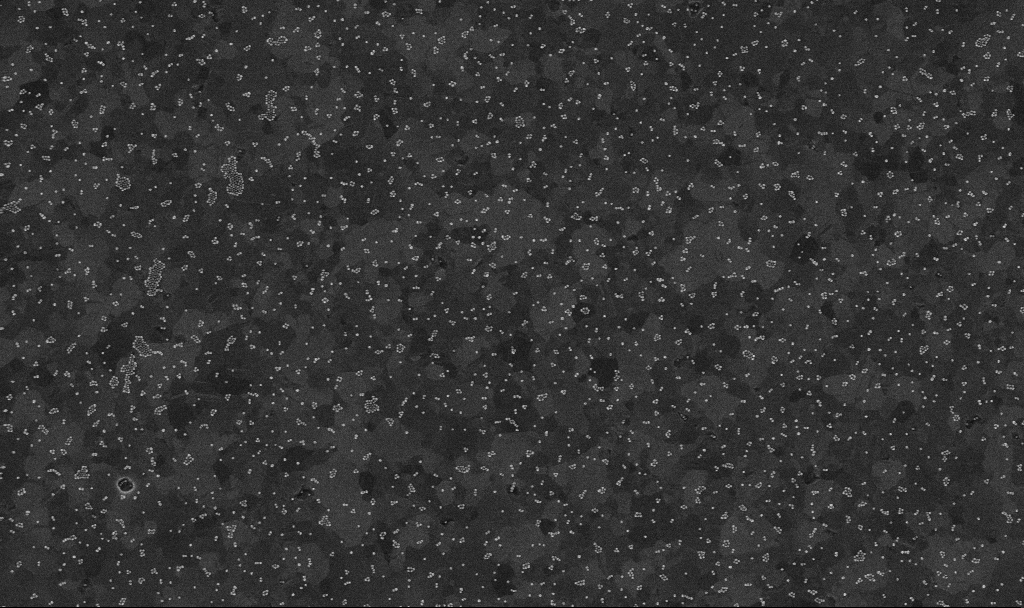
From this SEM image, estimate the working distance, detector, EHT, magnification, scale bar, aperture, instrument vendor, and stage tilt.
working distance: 3.4 mm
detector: InLens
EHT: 10 kV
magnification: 50 K X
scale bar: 1000 nm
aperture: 30 µm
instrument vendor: Zeiss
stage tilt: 0°